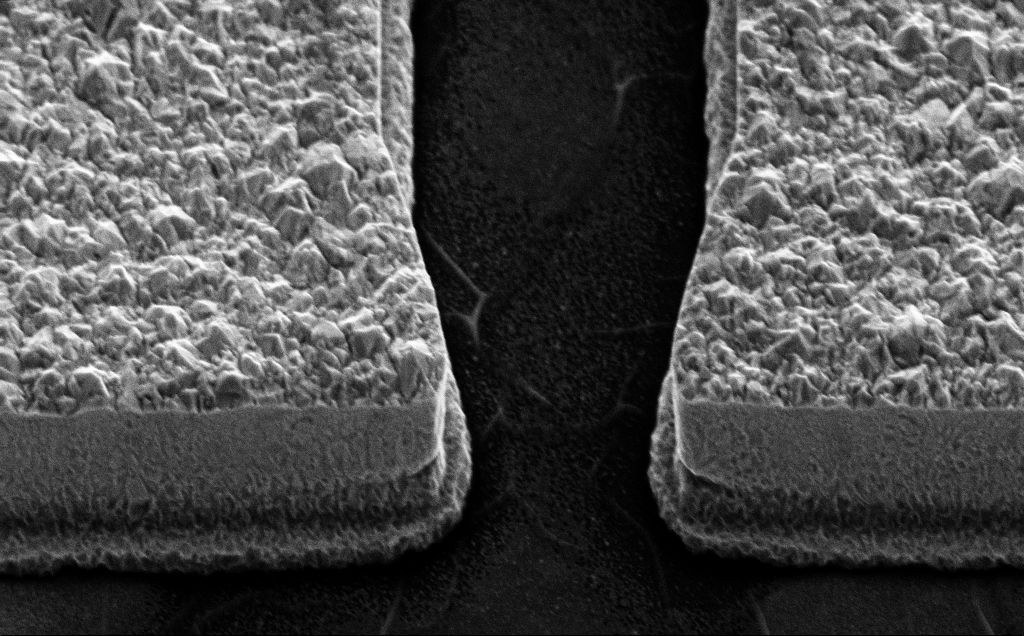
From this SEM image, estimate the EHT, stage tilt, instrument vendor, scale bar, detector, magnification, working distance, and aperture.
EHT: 10 kV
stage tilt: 45°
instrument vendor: Zeiss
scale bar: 2000 nm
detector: SE2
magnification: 13.23 K X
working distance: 15 mm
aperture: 30 µm